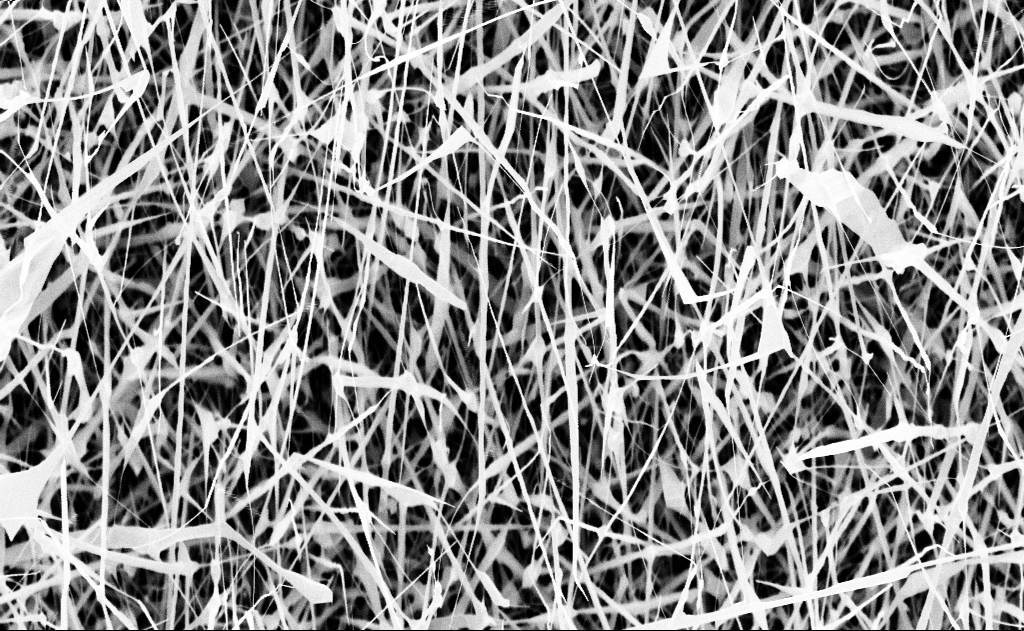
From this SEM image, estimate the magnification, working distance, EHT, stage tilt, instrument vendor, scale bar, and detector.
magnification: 20 K X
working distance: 16 mm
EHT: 10 kV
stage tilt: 0°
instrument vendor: Zeiss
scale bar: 1000 nm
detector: InLens